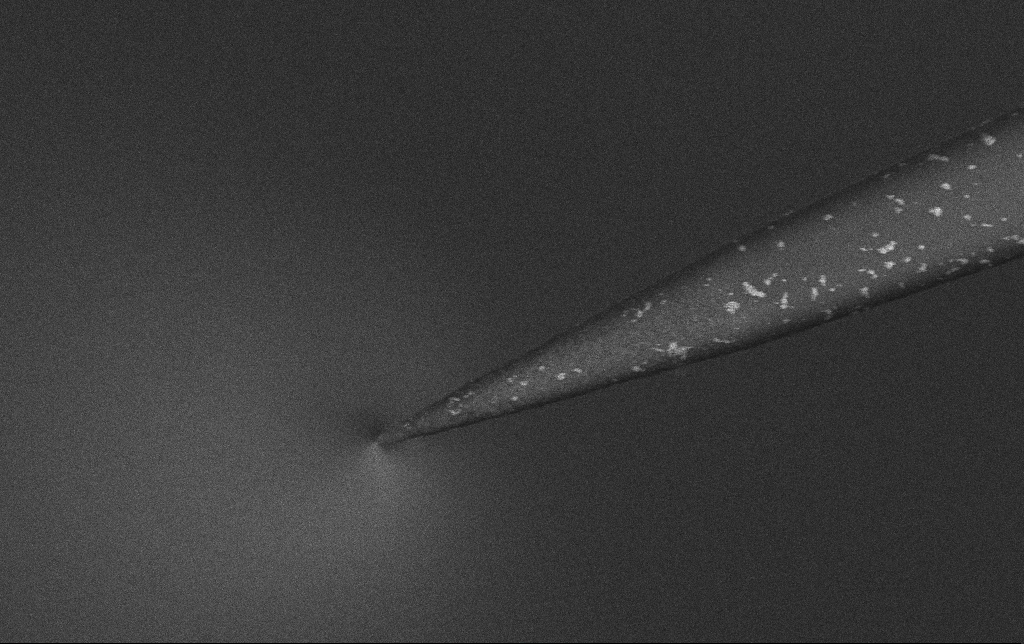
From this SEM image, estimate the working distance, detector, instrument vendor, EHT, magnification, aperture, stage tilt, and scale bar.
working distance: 6.6 mm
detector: InLens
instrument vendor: Zeiss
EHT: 3 kV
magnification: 25 K X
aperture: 30 µm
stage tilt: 0°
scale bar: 2000 nm